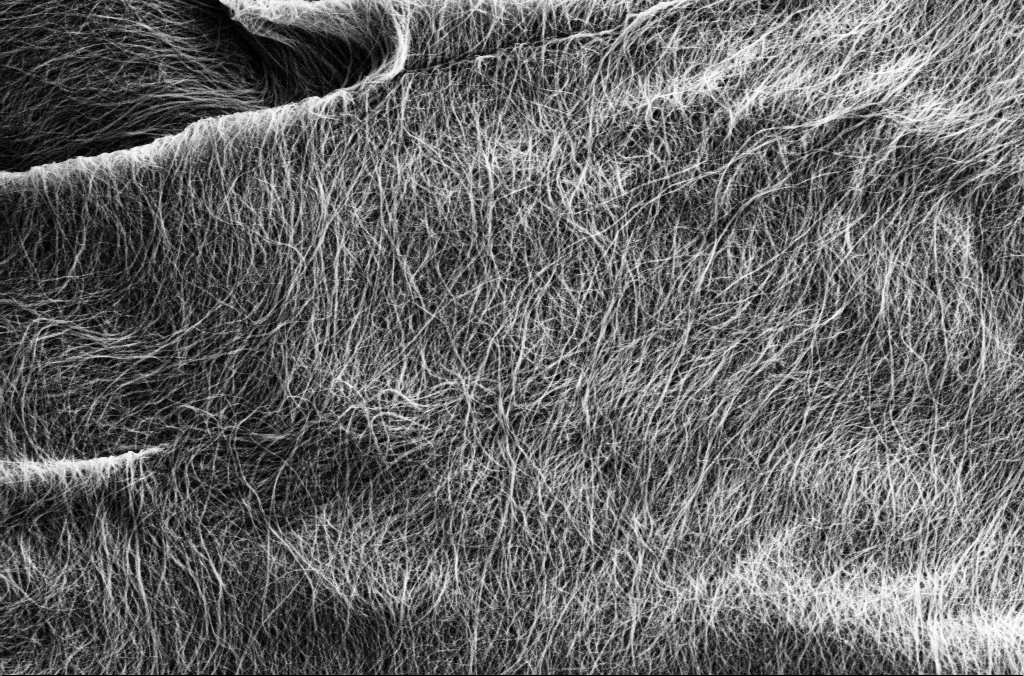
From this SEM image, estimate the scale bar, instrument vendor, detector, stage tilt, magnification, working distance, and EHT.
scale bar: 1000 nm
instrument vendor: Zeiss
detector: SE2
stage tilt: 0°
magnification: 25 K X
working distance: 5.7 mm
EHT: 1.8 kV